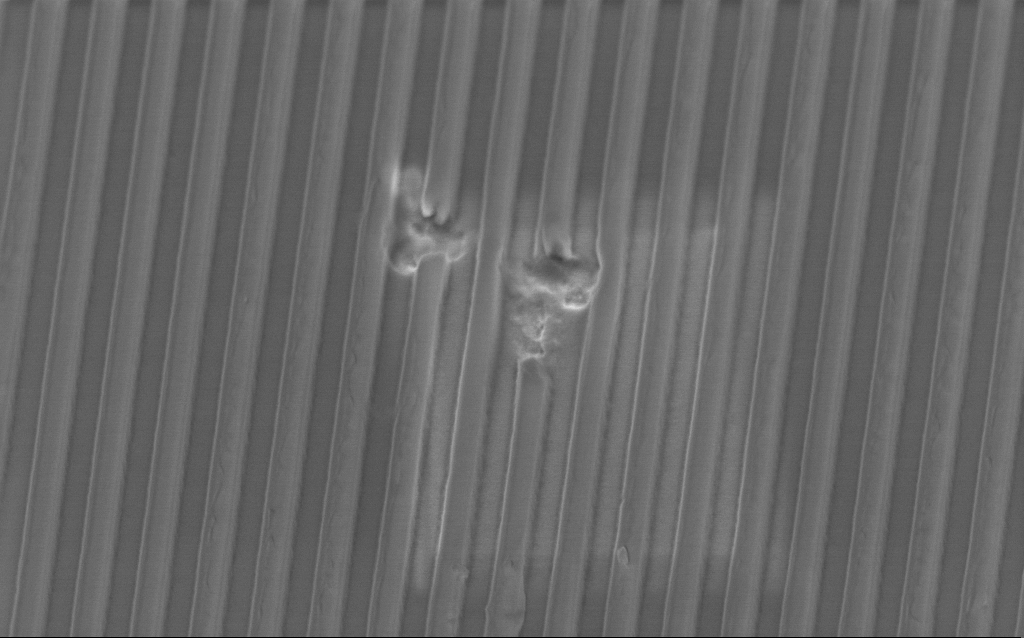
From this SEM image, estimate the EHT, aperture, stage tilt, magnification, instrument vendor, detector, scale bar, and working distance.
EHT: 2 kV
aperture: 30 µm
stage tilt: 45°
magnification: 43.3 K X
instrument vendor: Zeiss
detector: InLens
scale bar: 1000 nm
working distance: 3.6 mm